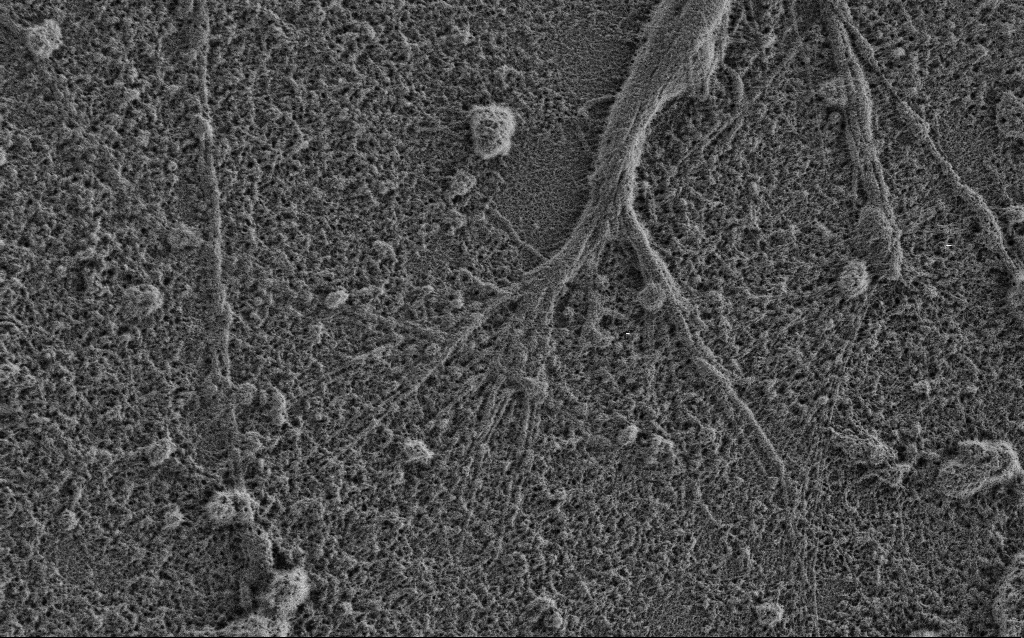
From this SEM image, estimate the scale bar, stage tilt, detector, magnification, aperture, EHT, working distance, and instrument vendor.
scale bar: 2000 nm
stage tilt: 0°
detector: SE2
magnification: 10 K X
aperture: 30 µm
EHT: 0.9 kV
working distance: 3.4 mm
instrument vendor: Zeiss